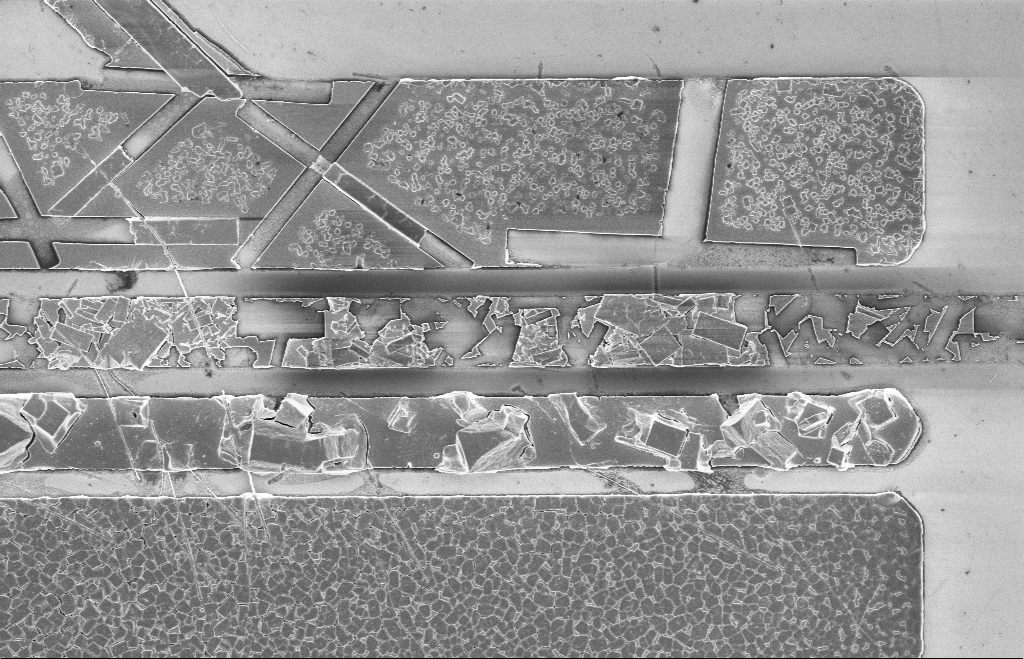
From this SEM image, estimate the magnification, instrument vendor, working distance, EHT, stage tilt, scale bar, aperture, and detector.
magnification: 6.21 K X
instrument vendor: Zeiss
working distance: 8 mm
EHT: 5 kV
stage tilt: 0°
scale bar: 2000 nm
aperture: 20 µm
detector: InLens